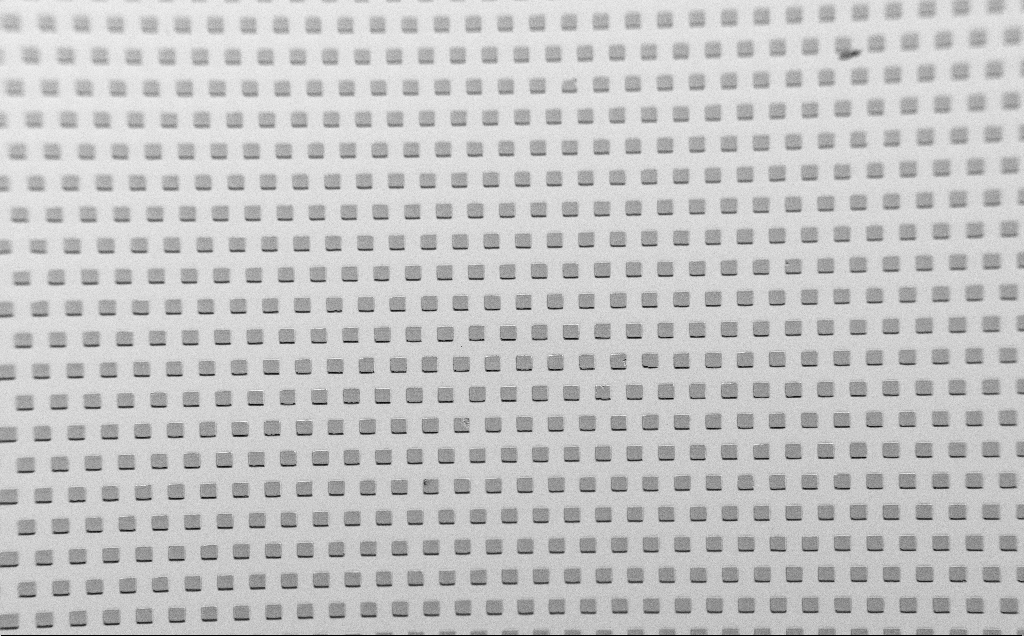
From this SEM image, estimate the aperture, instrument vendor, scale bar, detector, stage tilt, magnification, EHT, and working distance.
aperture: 30 µm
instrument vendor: Zeiss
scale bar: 100000 nm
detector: SE2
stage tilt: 45°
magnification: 0.291 K X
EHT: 1.5 kV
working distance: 7 mm